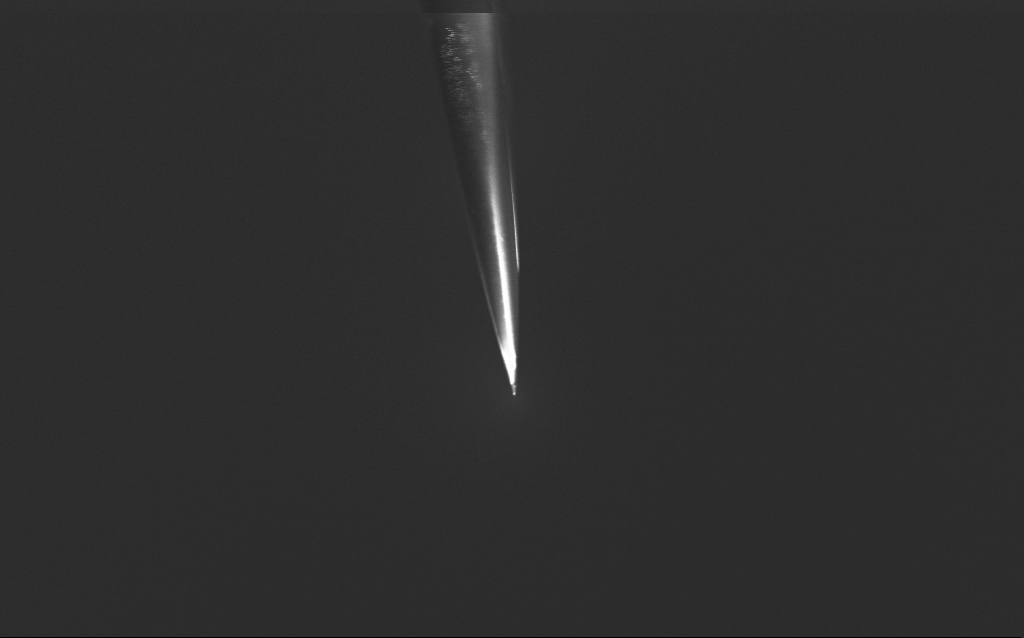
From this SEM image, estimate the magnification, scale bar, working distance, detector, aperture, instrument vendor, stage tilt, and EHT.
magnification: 10 K X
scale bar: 2000 nm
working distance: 5 mm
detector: InLens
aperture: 30 µm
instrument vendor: Zeiss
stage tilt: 45°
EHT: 2.5 kV